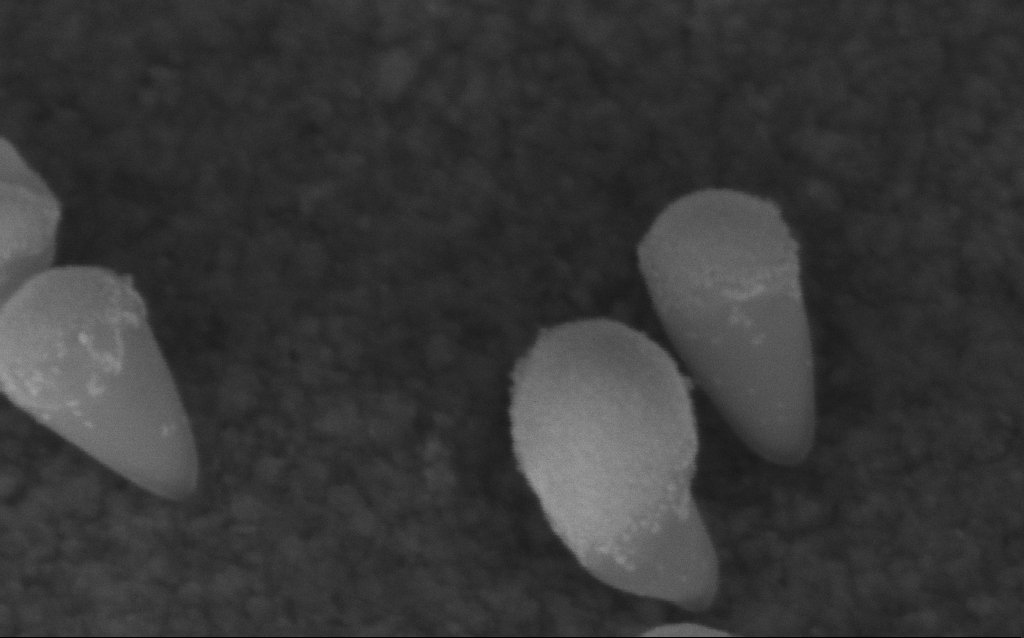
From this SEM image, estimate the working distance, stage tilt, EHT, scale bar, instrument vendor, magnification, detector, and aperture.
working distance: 6.3 mm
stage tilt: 45°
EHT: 5 kV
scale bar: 100 nm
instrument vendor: Zeiss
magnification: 394.35 K X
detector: InLens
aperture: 30 µm